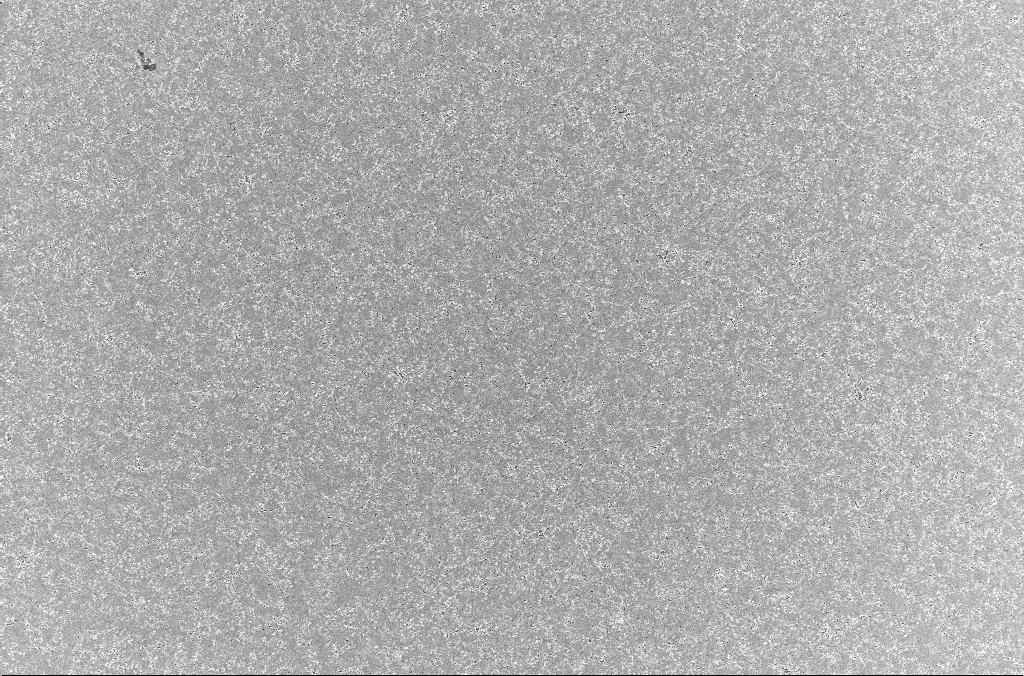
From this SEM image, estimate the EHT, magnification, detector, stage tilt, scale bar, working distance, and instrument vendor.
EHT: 5 kV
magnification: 1 K X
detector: InLens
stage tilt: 0°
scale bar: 20000 nm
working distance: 3.1 mm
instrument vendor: Zeiss